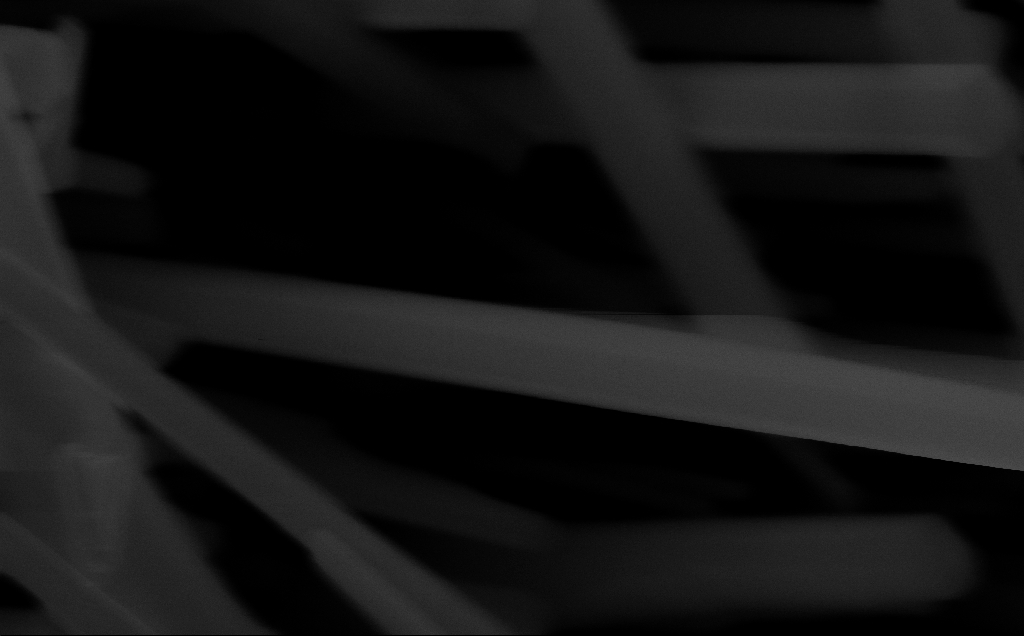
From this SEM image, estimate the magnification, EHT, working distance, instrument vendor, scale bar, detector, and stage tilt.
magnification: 188.51 K X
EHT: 10 kV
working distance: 6 mm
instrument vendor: Zeiss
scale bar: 200 nm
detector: InLens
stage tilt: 0°